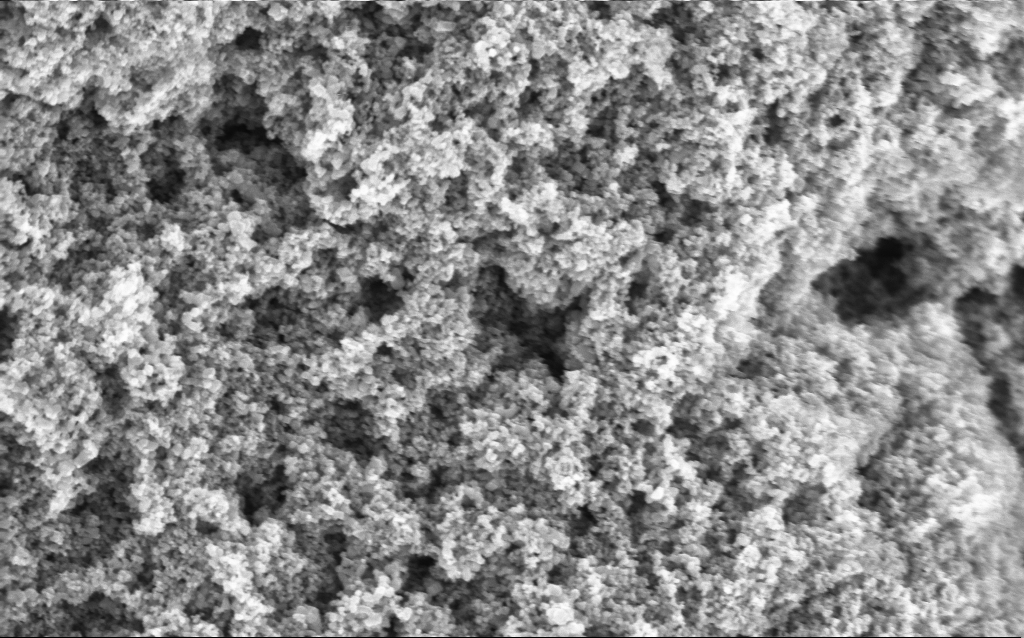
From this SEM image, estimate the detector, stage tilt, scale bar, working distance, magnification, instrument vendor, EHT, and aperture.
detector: InLens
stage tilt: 0°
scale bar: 1000 nm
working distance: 4.5 mm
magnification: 68.64 K X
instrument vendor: Zeiss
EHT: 5 kV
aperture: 30 µm